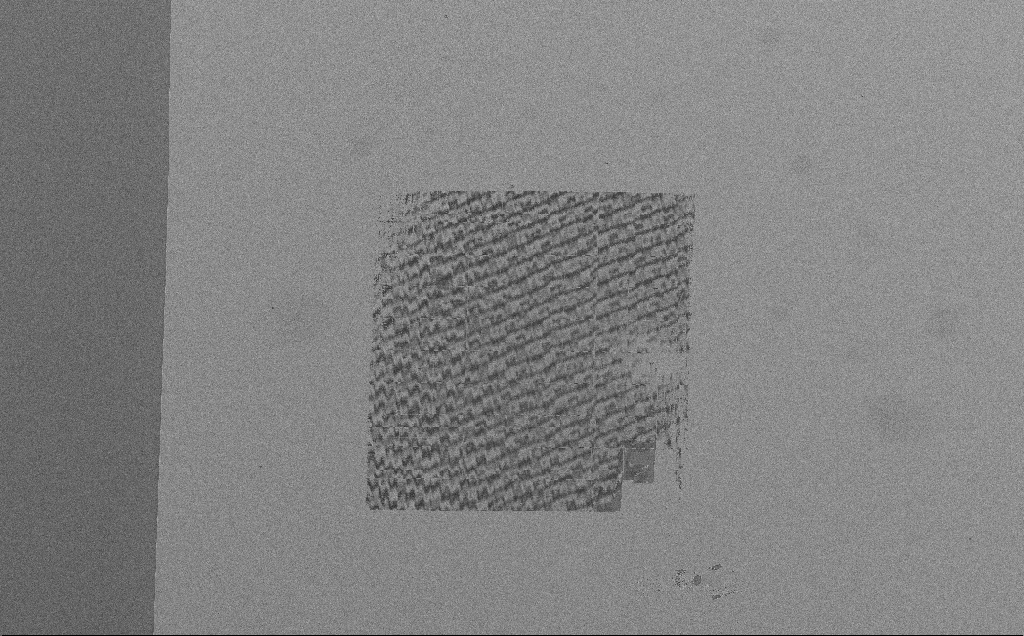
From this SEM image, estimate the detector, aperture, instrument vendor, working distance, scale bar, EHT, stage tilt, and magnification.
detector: SE2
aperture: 30 µm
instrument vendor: Zeiss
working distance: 2.3 mm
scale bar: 100000 nm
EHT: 5 kV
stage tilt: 0°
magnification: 0.395 K X